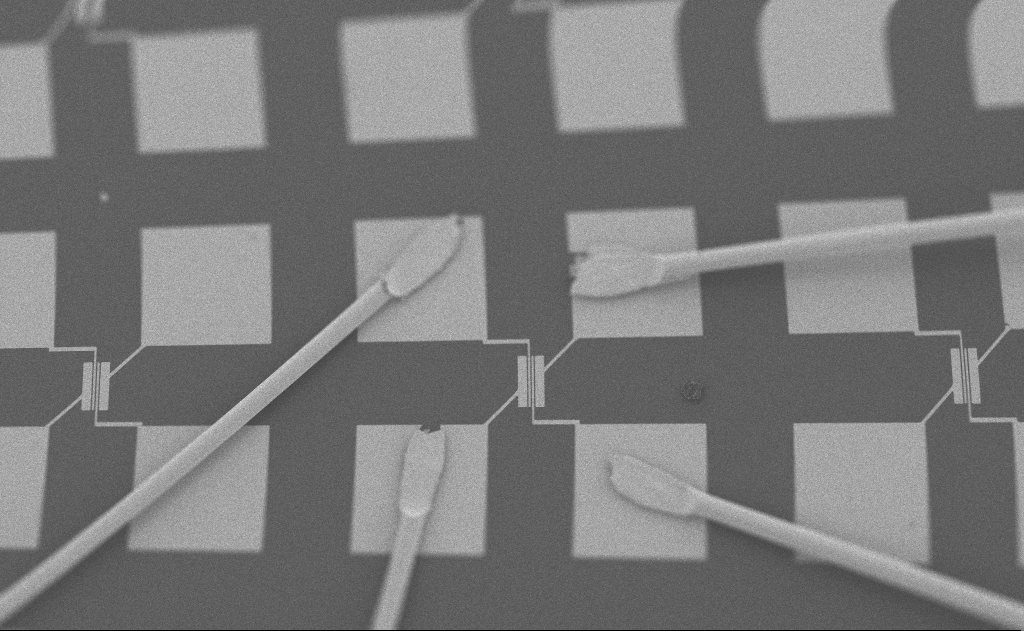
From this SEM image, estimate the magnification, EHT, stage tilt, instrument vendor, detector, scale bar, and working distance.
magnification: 0.323 K X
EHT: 5 kV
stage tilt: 0°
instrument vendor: Zeiss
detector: SE2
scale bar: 200000 nm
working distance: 10 mm